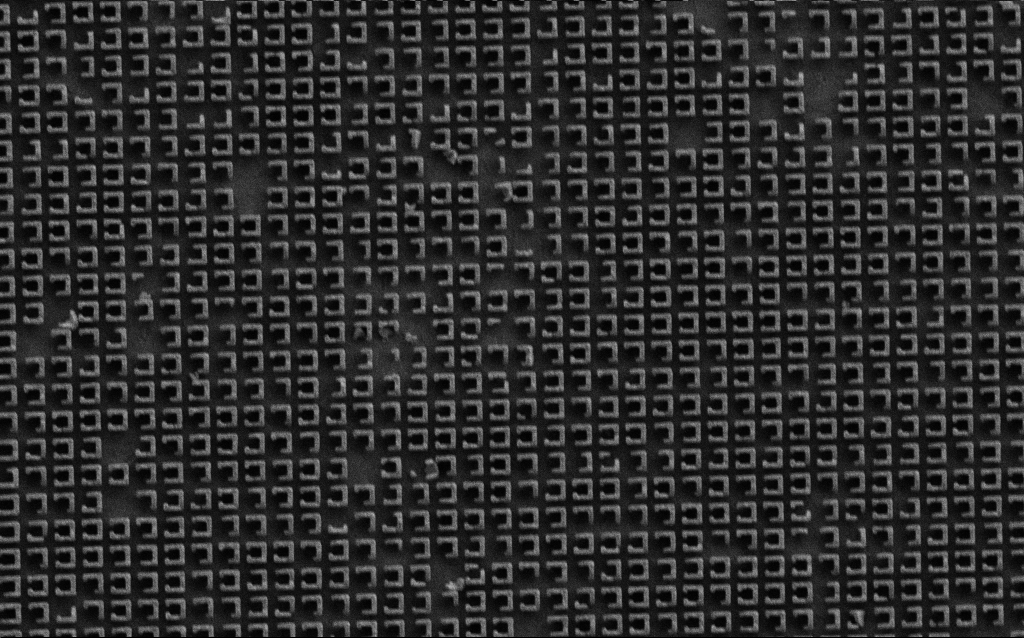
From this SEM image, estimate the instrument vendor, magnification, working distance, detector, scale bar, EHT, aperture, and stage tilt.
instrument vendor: Zeiss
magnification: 21.72 K X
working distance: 6.6 mm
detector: SE2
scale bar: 2000 nm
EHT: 3 kV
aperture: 30 µm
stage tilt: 0°